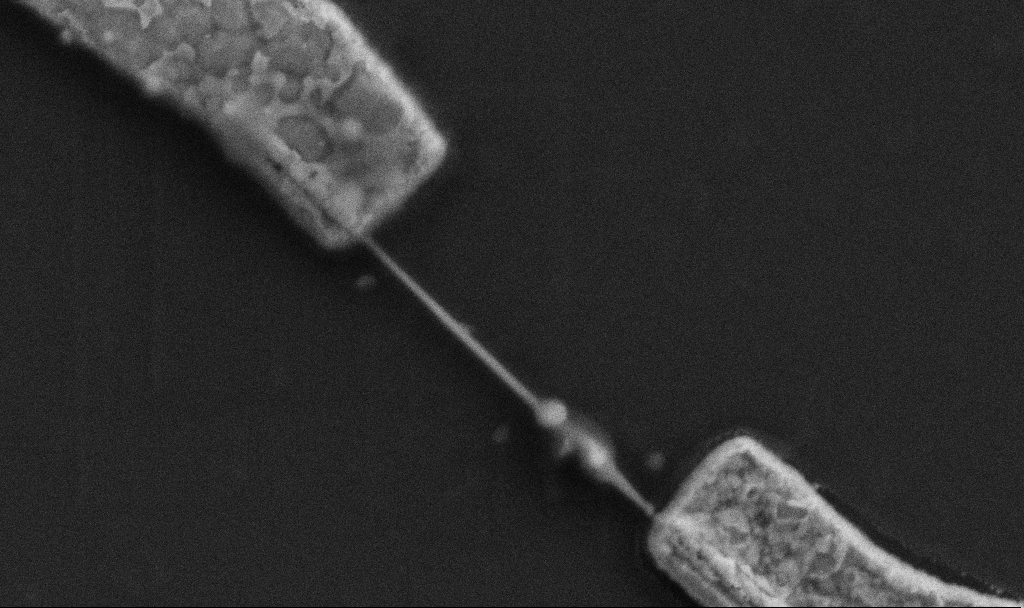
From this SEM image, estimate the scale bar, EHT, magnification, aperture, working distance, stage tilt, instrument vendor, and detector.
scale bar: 1000 nm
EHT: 5 kV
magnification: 50 K X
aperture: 30 µm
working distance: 8.5 mm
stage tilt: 0°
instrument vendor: Zeiss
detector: SE2